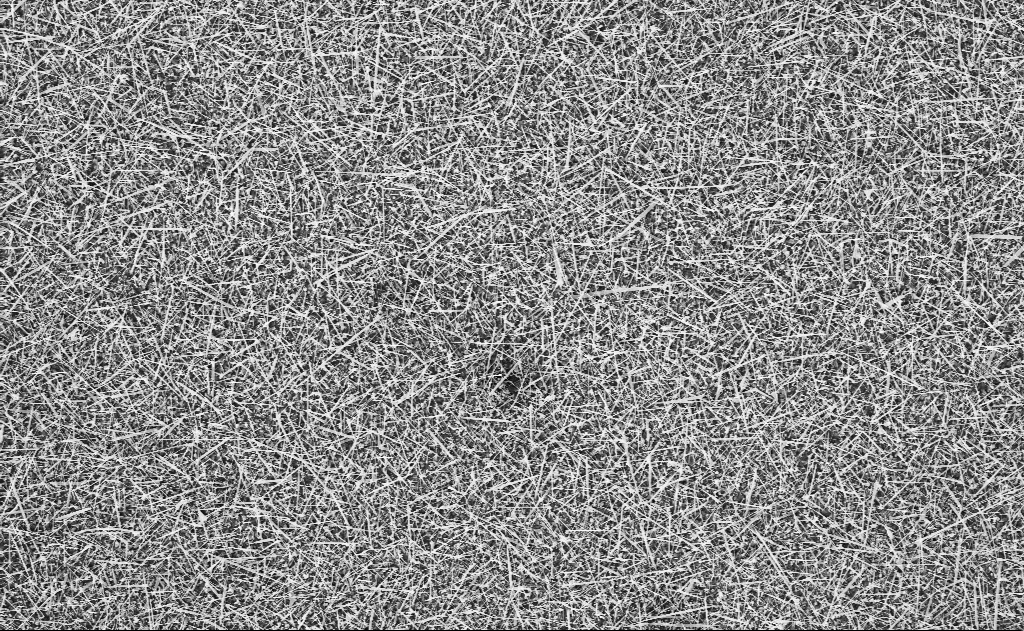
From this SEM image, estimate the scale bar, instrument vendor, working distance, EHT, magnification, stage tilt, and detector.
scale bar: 10000 nm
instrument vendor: Zeiss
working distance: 20 mm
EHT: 10 kV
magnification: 5 K X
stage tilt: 0°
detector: InLens